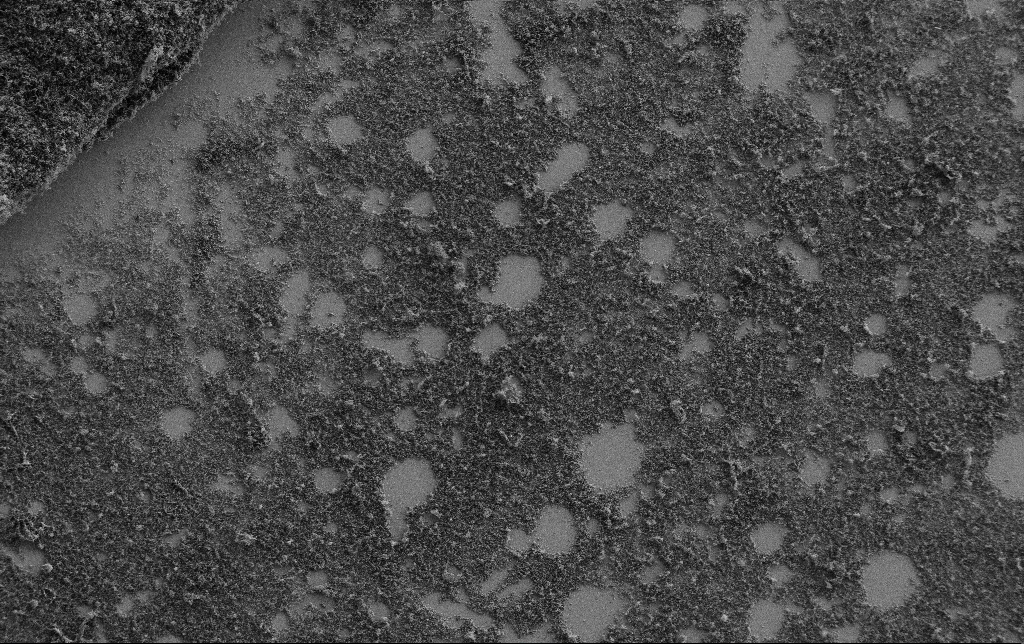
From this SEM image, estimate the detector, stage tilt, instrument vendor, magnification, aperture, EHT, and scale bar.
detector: SE2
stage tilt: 0°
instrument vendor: Zeiss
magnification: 0.5 K X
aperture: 30 µm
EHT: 2 kV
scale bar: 100000 nm